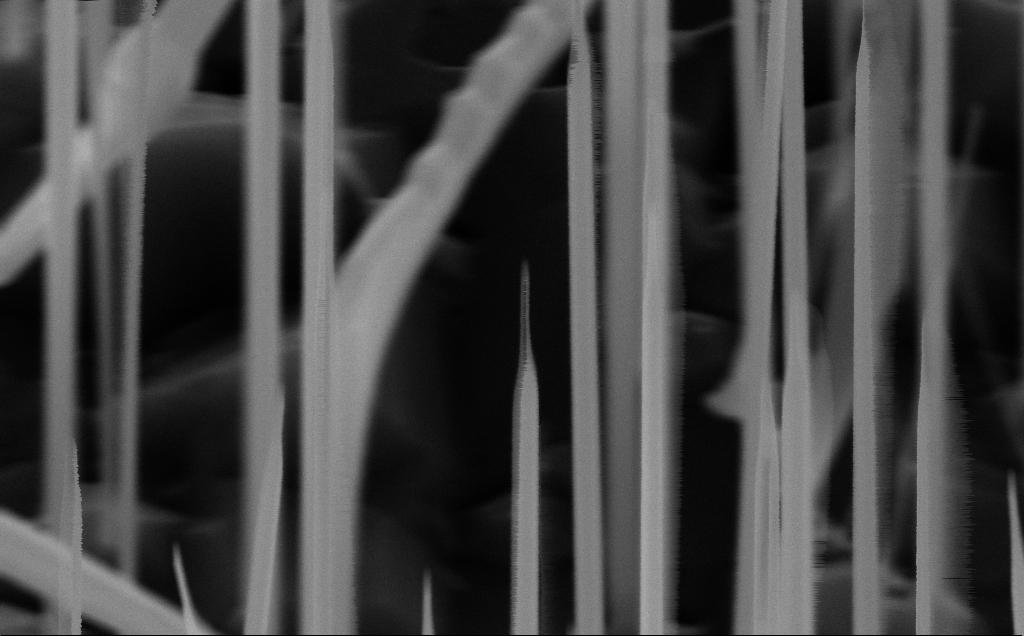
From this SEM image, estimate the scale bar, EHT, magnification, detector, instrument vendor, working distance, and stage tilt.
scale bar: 200 nm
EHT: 10 kV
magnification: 147.23 K X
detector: InLens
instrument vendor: Zeiss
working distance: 8 mm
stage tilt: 45°